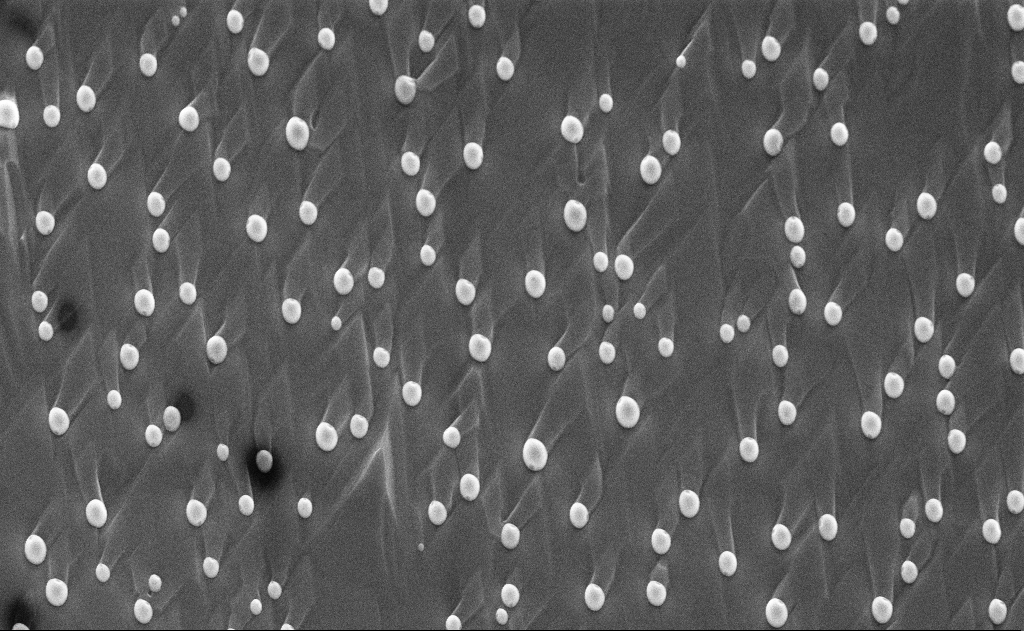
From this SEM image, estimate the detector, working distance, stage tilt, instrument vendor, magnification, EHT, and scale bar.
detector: InLens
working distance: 12 mm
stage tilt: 0°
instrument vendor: Zeiss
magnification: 10 K X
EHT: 10 kV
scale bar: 2000 nm